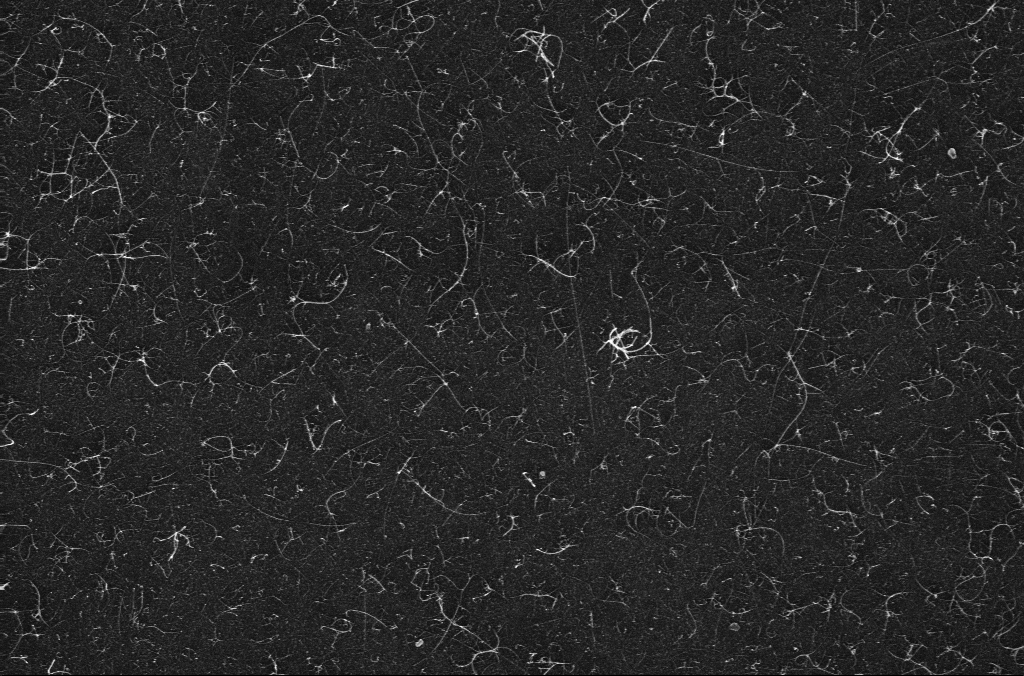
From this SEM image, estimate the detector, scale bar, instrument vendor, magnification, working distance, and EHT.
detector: InLens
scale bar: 1000 nm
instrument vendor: Zeiss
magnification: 45.35 K X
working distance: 3.3 mm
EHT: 10 kV